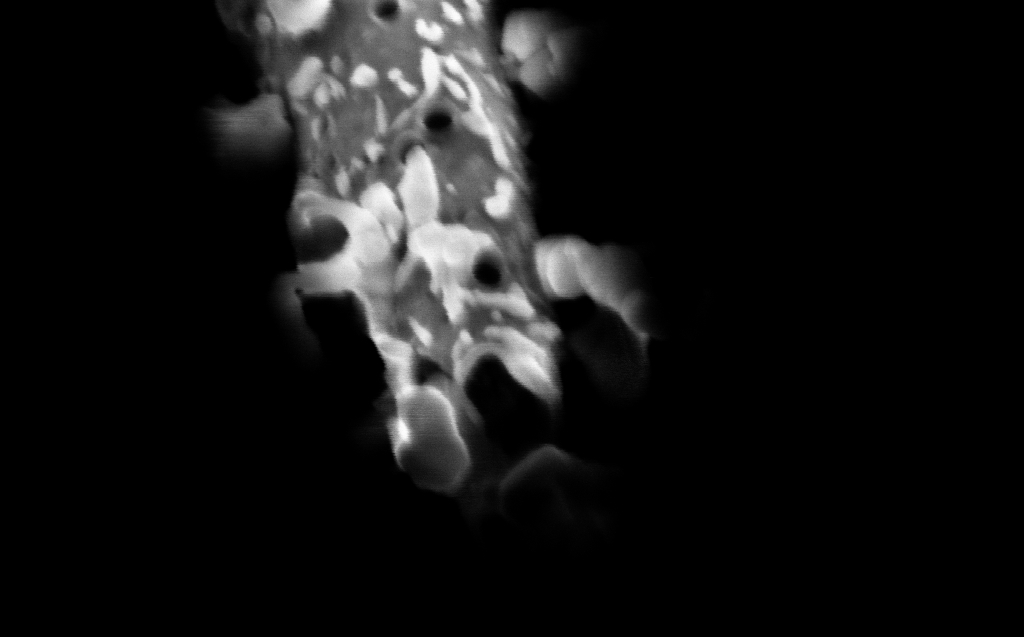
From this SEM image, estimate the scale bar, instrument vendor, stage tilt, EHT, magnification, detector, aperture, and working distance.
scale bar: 200 nm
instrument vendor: Zeiss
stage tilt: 45°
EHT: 5 kV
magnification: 200.31 K X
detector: InLens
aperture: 30 µm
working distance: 4 mm